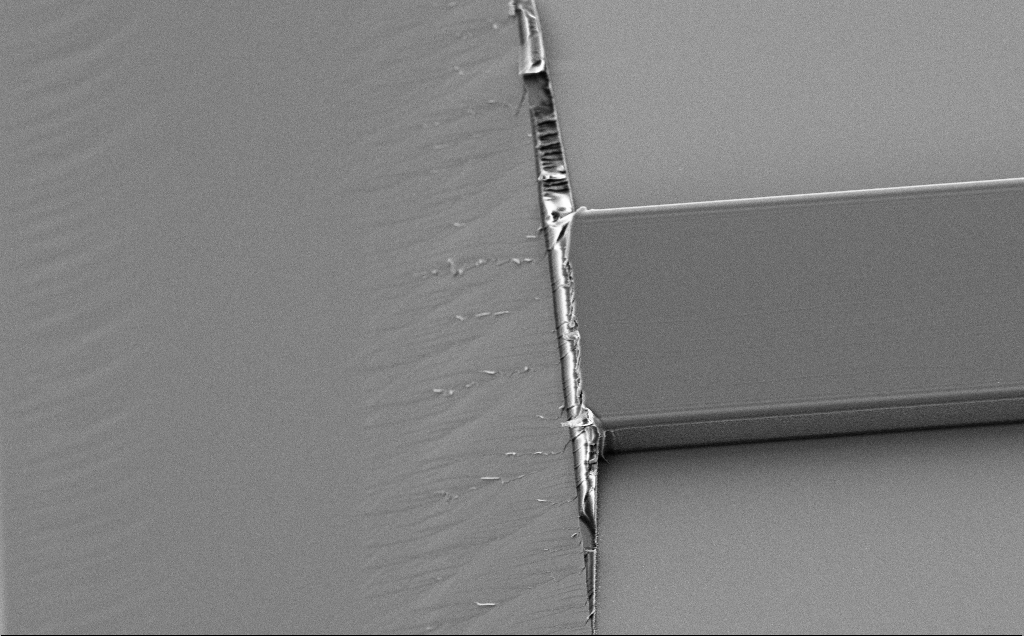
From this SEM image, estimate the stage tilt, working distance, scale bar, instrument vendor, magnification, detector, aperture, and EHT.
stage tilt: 30°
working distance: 11 mm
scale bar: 20000 nm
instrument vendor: Zeiss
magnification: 1.91 K X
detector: SE2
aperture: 30 µm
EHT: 5 kV